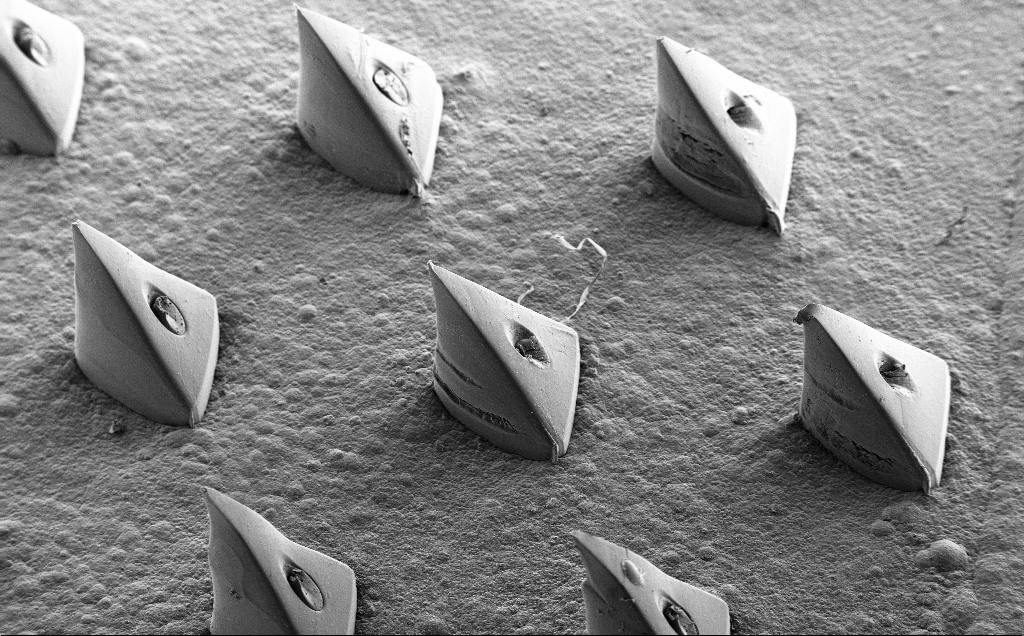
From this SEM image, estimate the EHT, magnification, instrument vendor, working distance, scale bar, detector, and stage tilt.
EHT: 10 kV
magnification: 0.091 K X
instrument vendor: Zeiss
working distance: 10 mm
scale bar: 200000 nm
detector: SE2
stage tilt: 35.3°